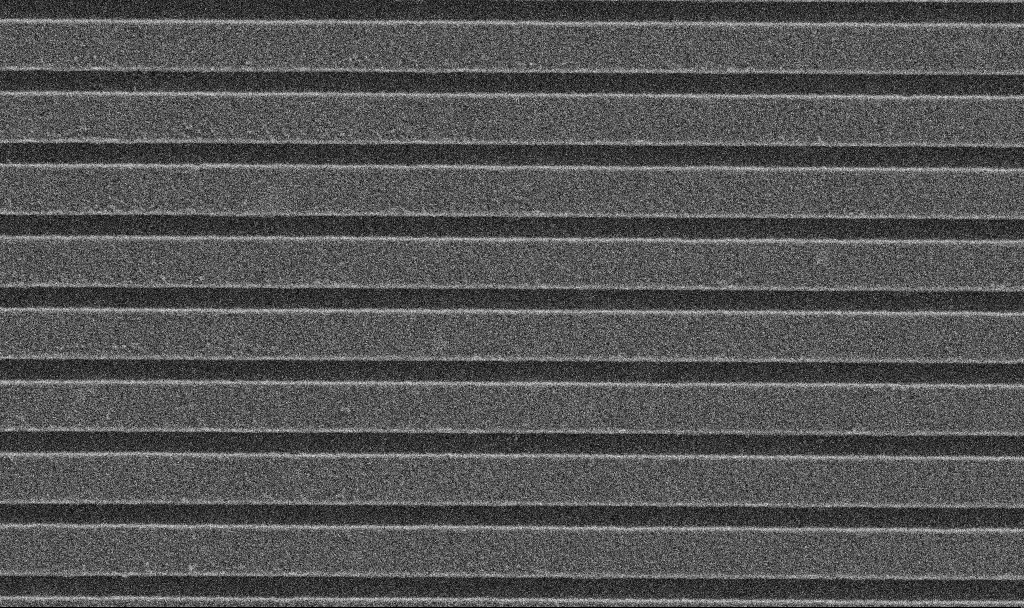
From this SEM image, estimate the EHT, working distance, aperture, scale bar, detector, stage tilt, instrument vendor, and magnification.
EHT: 5 kV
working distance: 2.9 mm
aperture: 30 µm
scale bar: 1000 nm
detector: SE2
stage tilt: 0°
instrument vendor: Zeiss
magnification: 33.34 K X